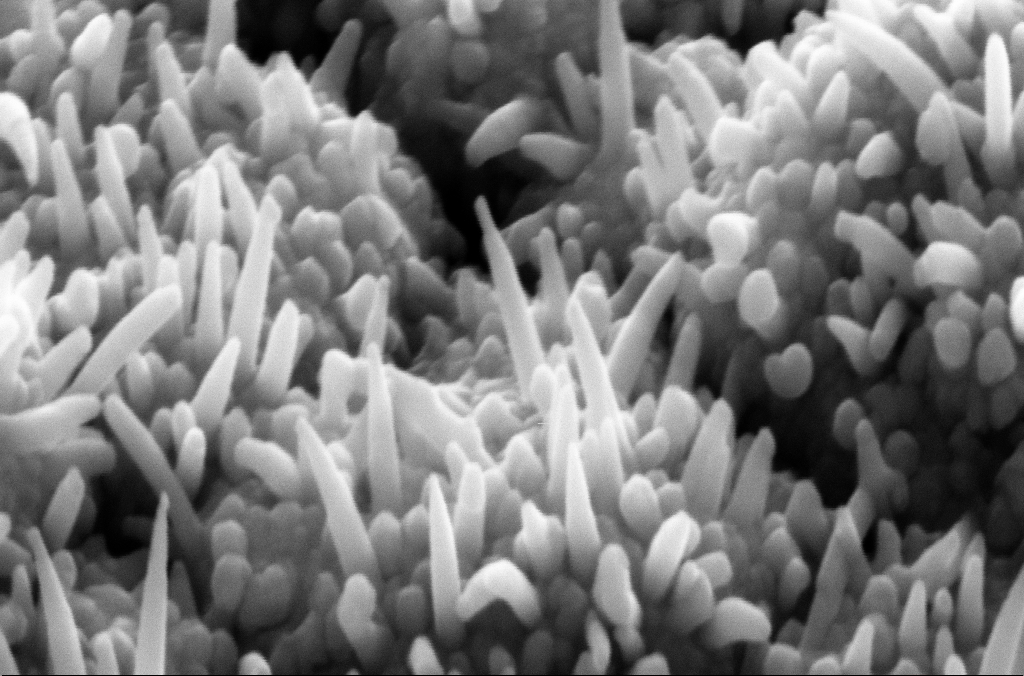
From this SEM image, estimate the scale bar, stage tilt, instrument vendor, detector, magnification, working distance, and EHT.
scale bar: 200 nm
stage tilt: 45°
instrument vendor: Zeiss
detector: SE2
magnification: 167.27 K X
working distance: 8 mm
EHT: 10 kV